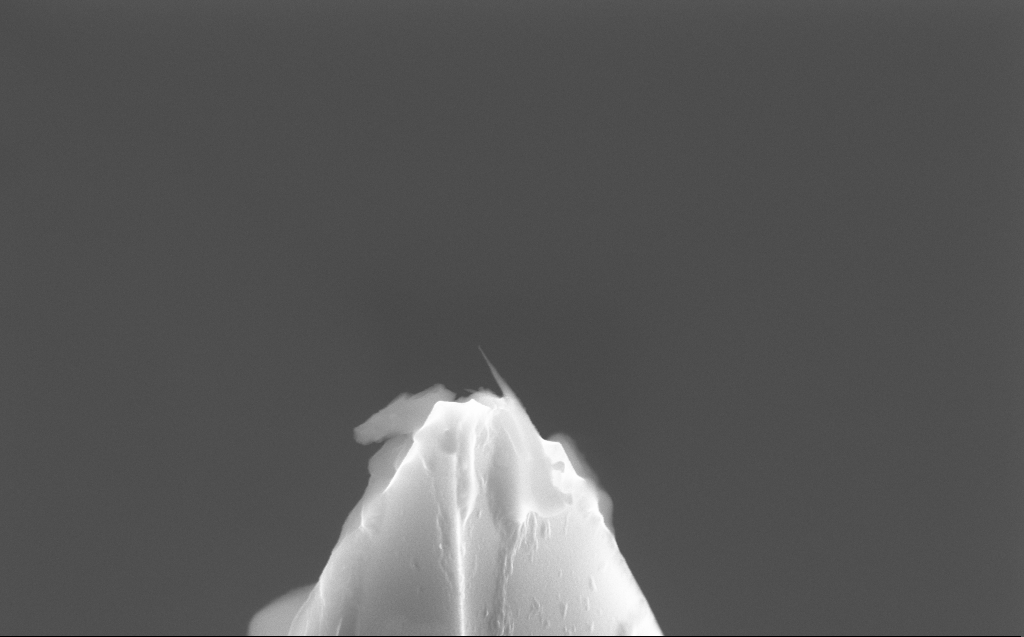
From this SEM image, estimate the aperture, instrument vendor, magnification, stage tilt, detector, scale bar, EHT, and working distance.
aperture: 30 µm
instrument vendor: Zeiss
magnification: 58.03 K X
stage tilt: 45°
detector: InLens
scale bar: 1000 nm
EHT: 10 kV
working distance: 3 mm